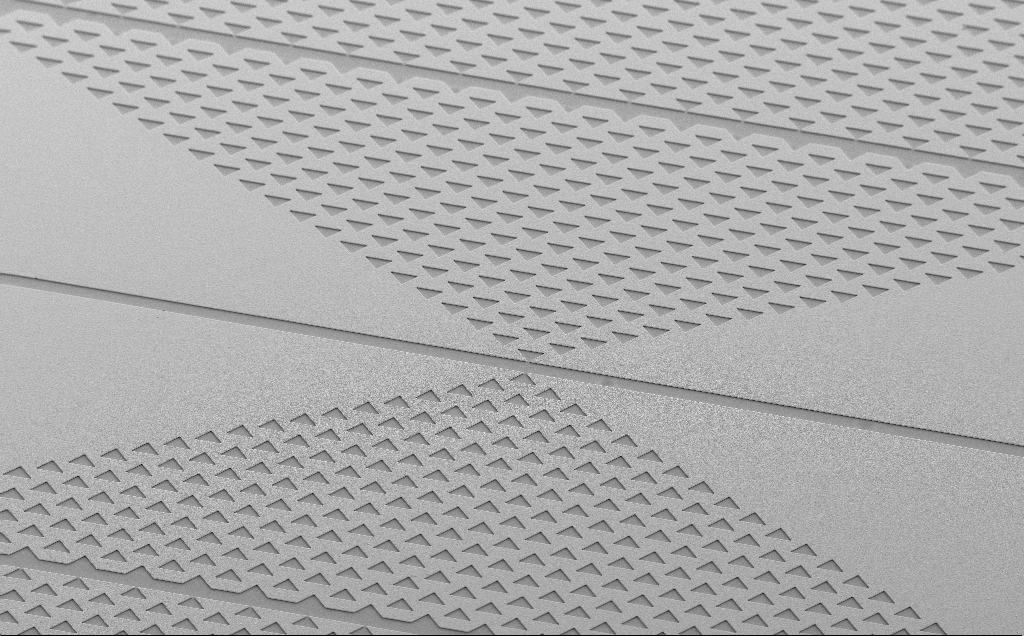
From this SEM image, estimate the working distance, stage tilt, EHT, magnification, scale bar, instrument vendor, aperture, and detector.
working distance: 13 mm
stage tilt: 35°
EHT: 5 kV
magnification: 0.275 K X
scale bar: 100000 nm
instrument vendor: Zeiss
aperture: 30 µm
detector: SE2